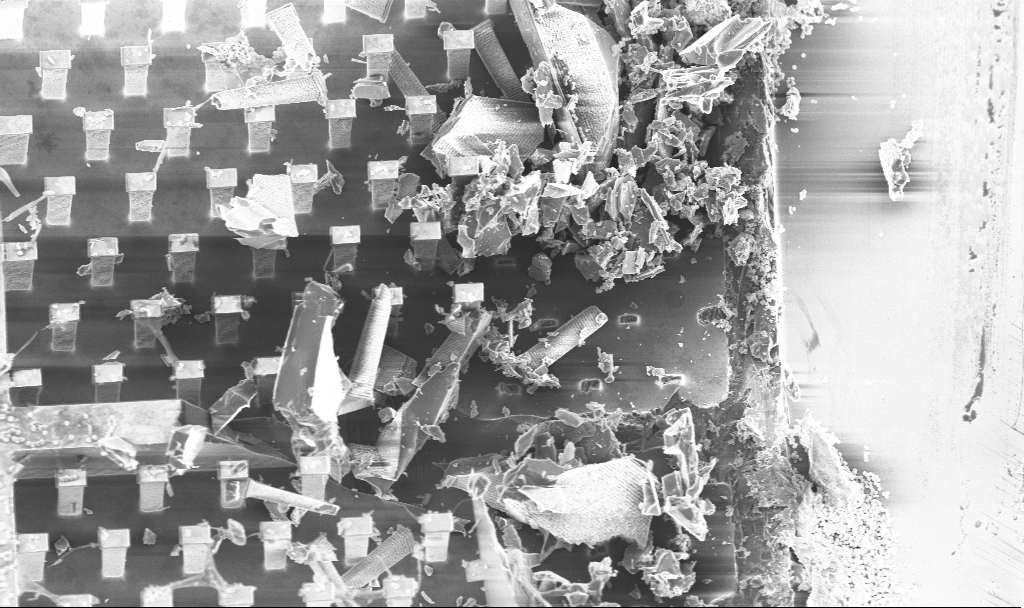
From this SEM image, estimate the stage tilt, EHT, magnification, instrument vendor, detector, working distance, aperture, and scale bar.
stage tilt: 20°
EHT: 5 kV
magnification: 2.46 K X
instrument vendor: Zeiss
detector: InLens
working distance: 3.3 mm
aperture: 30 µm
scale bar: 20000 nm